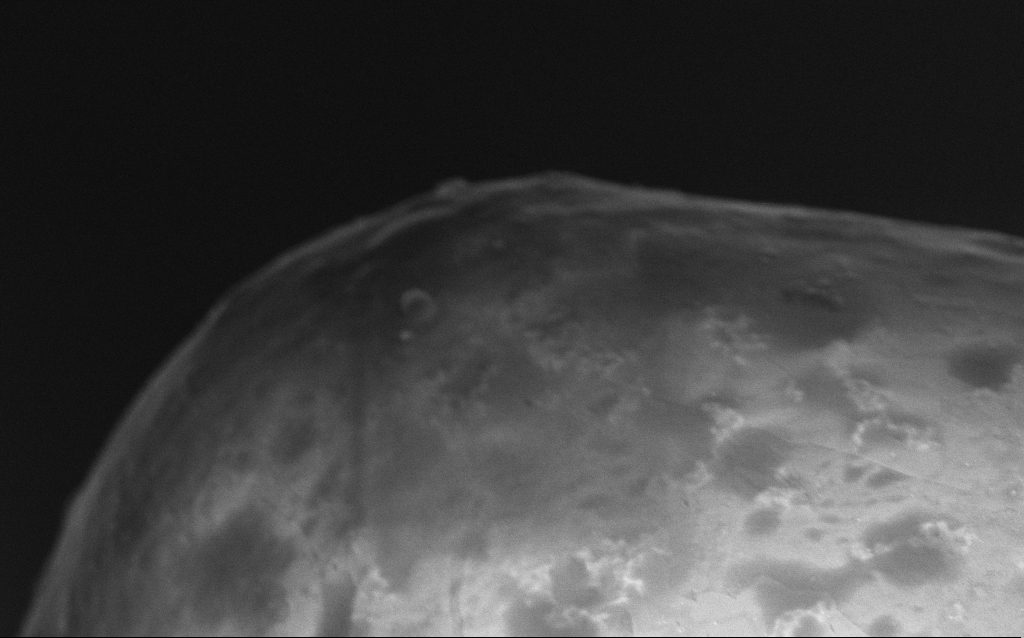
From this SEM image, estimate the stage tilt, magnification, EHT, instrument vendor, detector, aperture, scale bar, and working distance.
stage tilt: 0°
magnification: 67.82 K X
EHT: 5 kV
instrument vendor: Zeiss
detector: InLens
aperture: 30 µm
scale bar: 1000 nm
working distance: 3.1 mm